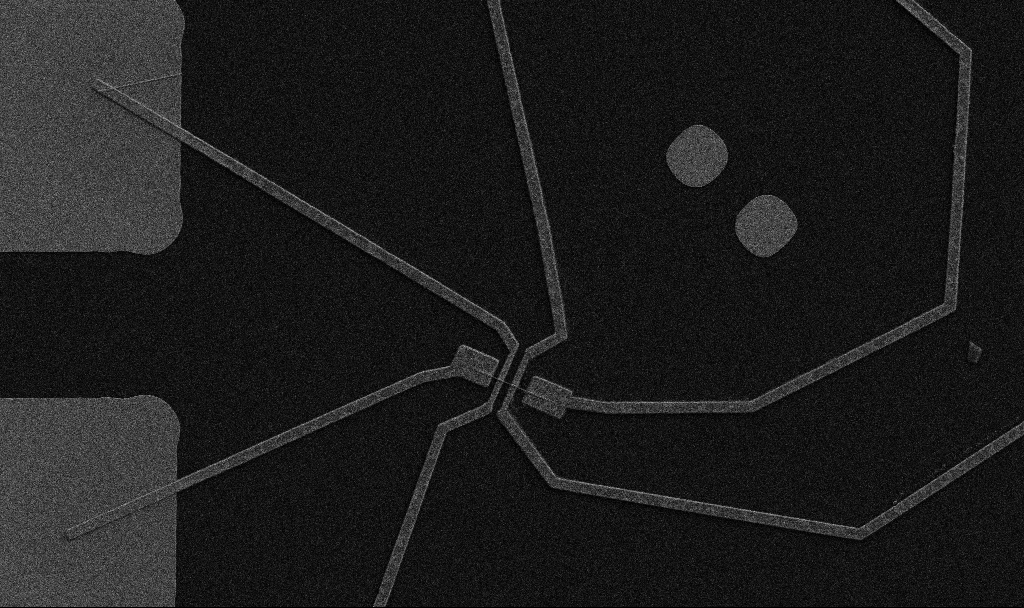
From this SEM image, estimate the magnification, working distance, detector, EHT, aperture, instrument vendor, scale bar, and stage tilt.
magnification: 5 K X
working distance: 8.7 mm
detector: SE2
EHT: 5 kV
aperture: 30 µm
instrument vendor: Zeiss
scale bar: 10000 nm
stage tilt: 0°